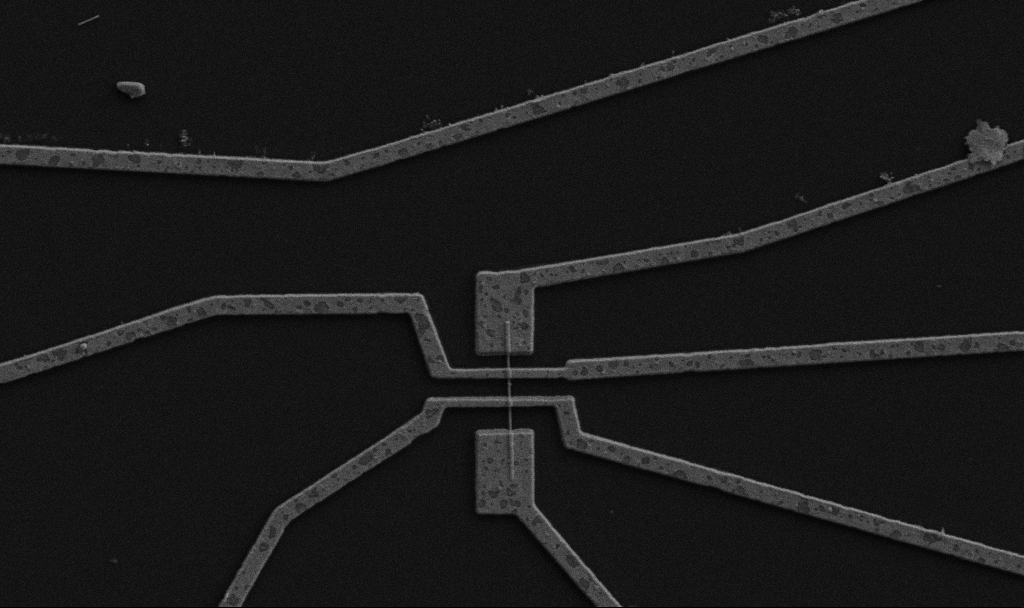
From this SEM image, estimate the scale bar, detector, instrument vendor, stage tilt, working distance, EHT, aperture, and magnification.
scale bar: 2000 nm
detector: SE2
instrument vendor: Zeiss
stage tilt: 0°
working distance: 9.7 mm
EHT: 5 kV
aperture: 30 µm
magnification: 10 K X